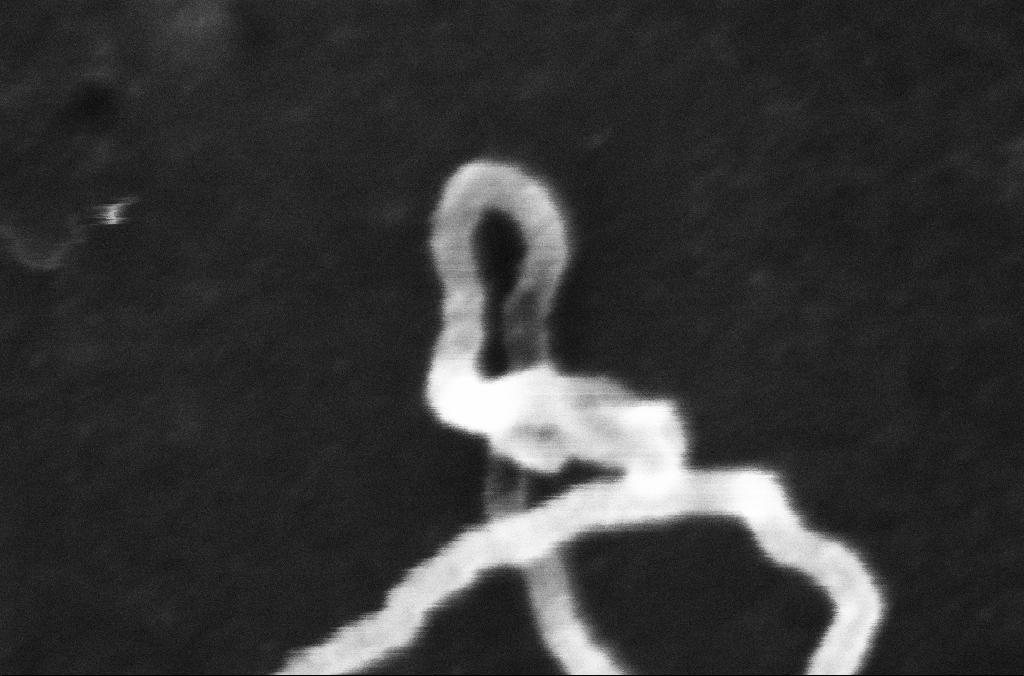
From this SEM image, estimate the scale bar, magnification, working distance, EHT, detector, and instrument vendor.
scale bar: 20 nm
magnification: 845.15 K X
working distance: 3.2 mm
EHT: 10 kV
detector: InLens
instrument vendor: Zeiss